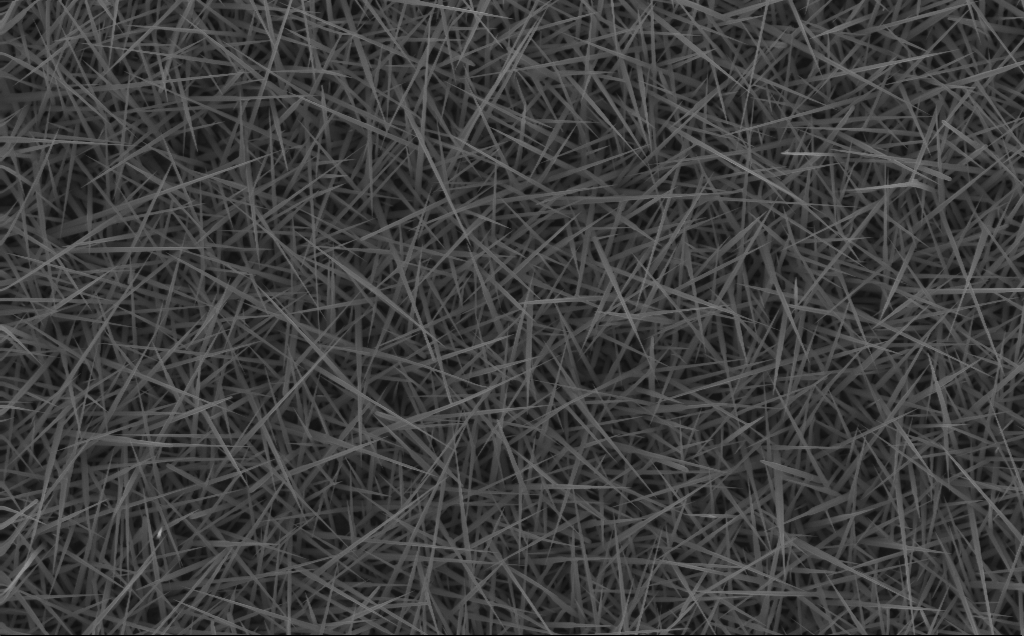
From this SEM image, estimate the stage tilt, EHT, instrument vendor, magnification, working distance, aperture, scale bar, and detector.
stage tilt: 0°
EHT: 10 kV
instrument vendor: Zeiss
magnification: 20 K X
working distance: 4 mm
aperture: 30 µm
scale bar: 2000 nm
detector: InLens